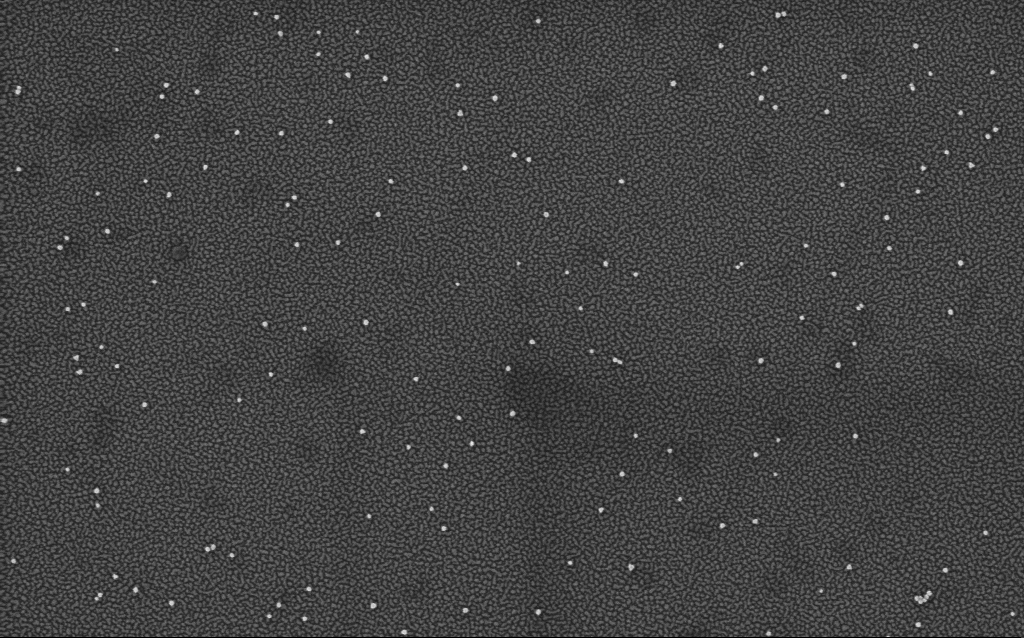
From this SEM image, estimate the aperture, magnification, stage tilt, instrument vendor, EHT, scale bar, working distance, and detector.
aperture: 30 µm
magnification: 100 K X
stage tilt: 0°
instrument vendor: Zeiss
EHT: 1 kV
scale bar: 200 nm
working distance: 1.7 mm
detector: InLens